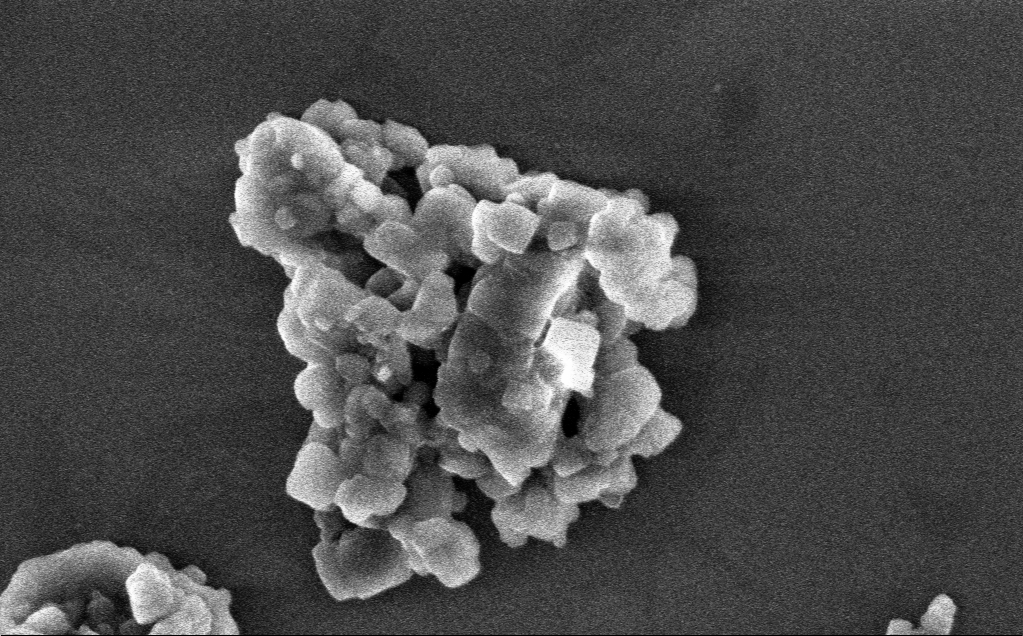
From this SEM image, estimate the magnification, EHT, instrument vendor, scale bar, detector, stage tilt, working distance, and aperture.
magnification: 124.47 K X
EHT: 3 kV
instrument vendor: Zeiss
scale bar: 200 nm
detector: InLens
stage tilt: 0°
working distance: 3 mm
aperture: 30 µm